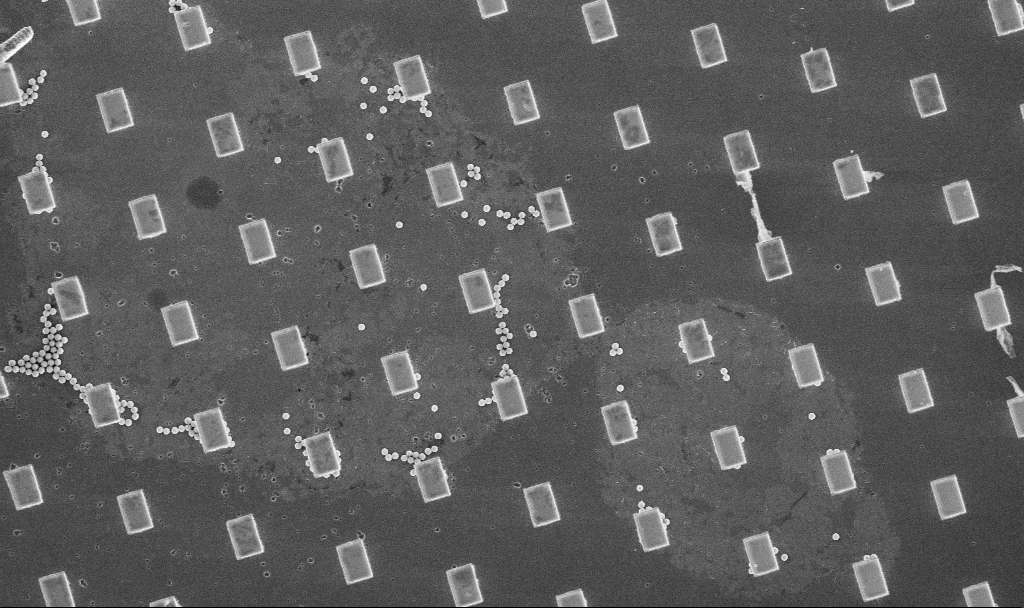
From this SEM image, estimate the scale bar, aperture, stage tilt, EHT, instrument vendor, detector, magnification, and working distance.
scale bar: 10000 nm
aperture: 30 µm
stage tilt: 0°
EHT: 5 kV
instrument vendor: Zeiss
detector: InLens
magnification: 3.43 K X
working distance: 4.9 mm